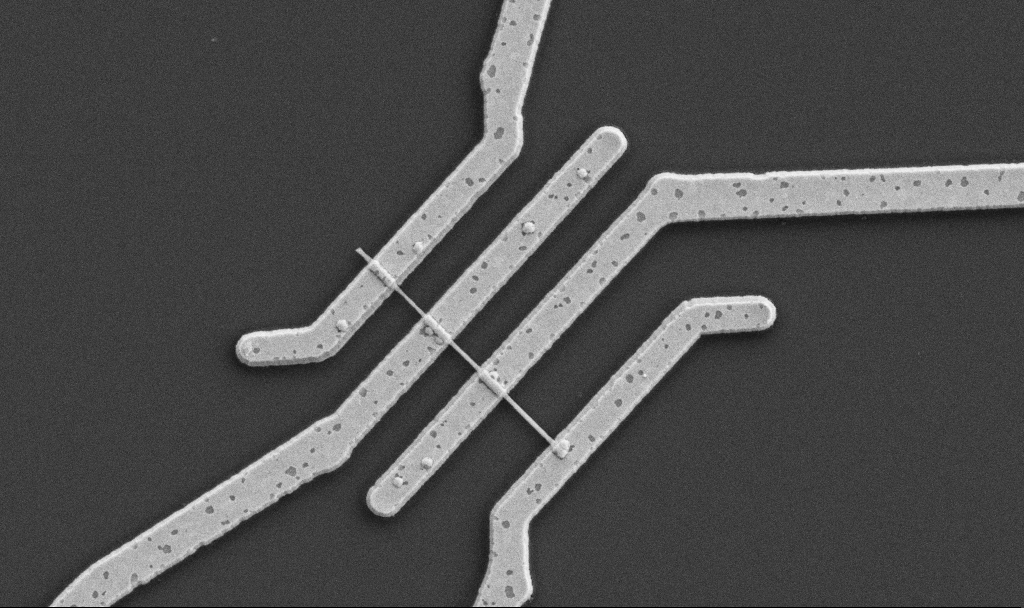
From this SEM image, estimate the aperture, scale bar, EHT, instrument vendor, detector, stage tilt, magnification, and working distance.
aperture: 30 µm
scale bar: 1000 nm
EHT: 5 kV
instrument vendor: Zeiss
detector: SE2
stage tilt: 0°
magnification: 20 K X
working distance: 10.7 mm